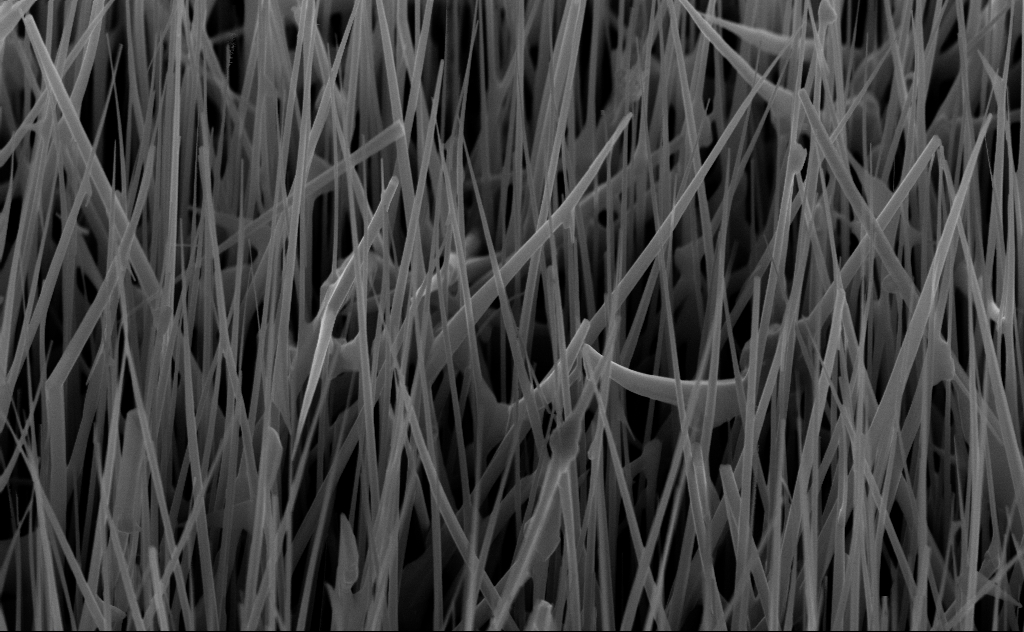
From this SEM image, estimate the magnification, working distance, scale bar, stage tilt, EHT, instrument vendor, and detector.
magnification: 40 K X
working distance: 6 mm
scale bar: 1000 nm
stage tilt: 45°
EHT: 10 kV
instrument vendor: Zeiss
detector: InLens